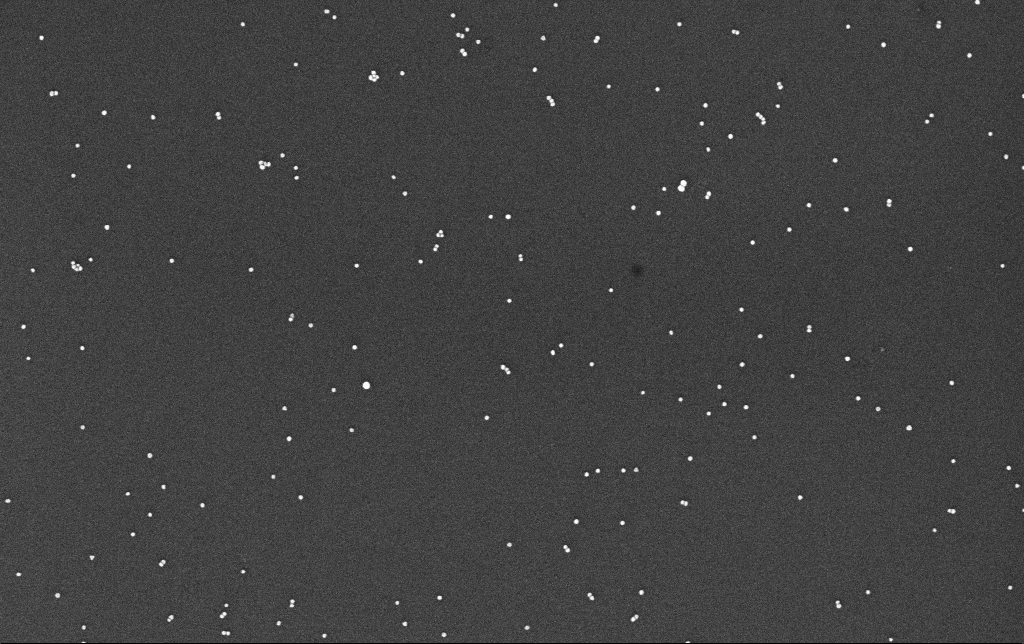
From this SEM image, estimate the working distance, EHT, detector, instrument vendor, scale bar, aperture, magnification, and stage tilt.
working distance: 3.3 mm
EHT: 10 kV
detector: InLens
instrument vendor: Zeiss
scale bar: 200 nm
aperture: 30 µm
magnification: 100 K X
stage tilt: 0°